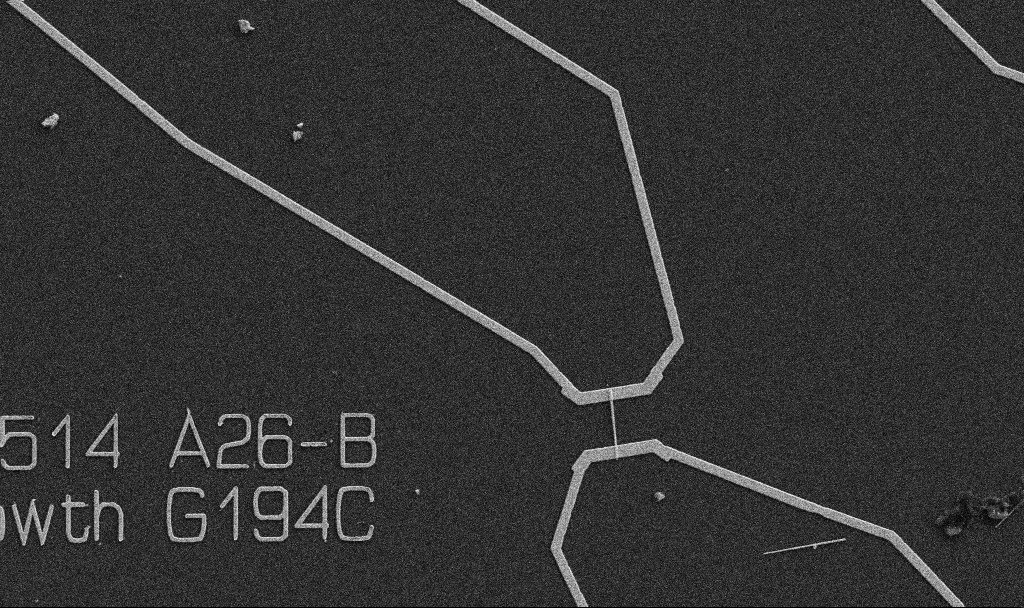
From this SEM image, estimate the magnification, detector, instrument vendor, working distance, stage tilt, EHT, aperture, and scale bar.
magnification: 5 K X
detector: SE2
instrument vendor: Zeiss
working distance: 10.7 mm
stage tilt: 0°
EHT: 5 kV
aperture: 30 µm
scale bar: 10000 nm